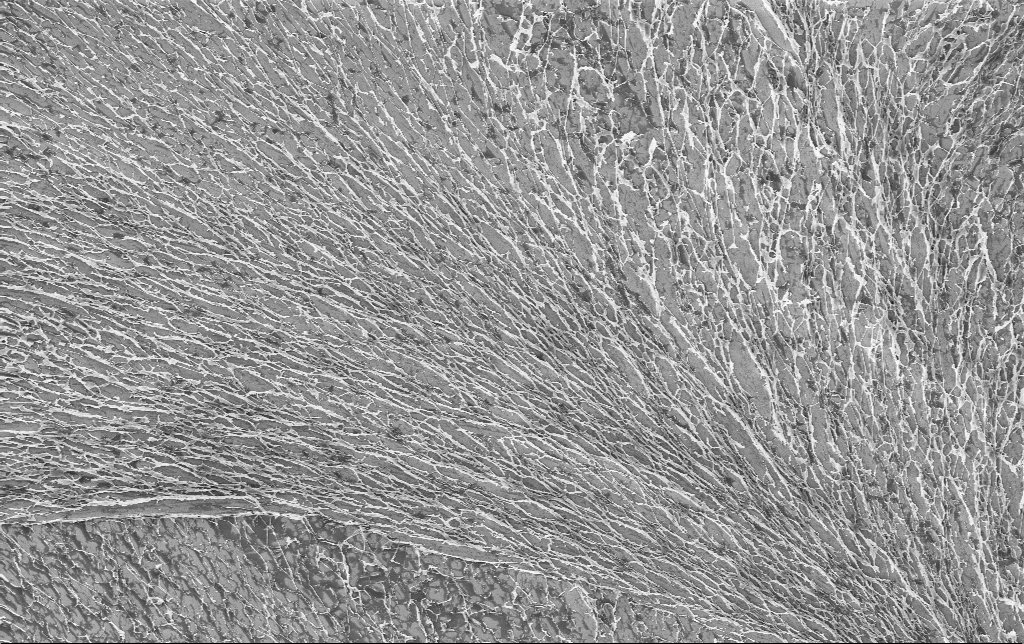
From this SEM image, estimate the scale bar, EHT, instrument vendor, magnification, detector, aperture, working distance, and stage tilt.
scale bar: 10000 nm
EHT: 3 kV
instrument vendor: Zeiss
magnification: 2.26 K X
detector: InLens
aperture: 30 µm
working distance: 3.4 mm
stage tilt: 0°